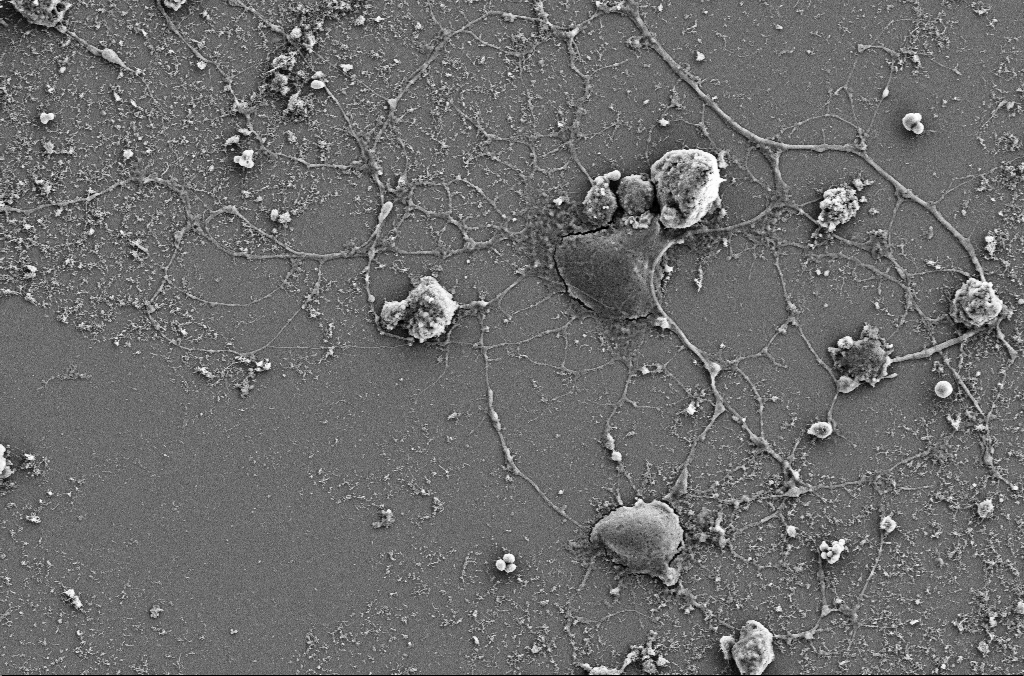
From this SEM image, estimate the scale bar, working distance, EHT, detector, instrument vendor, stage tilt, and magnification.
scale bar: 10000 nm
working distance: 4 mm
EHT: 5 kV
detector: SE2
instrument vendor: Zeiss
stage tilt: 0°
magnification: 4 K X